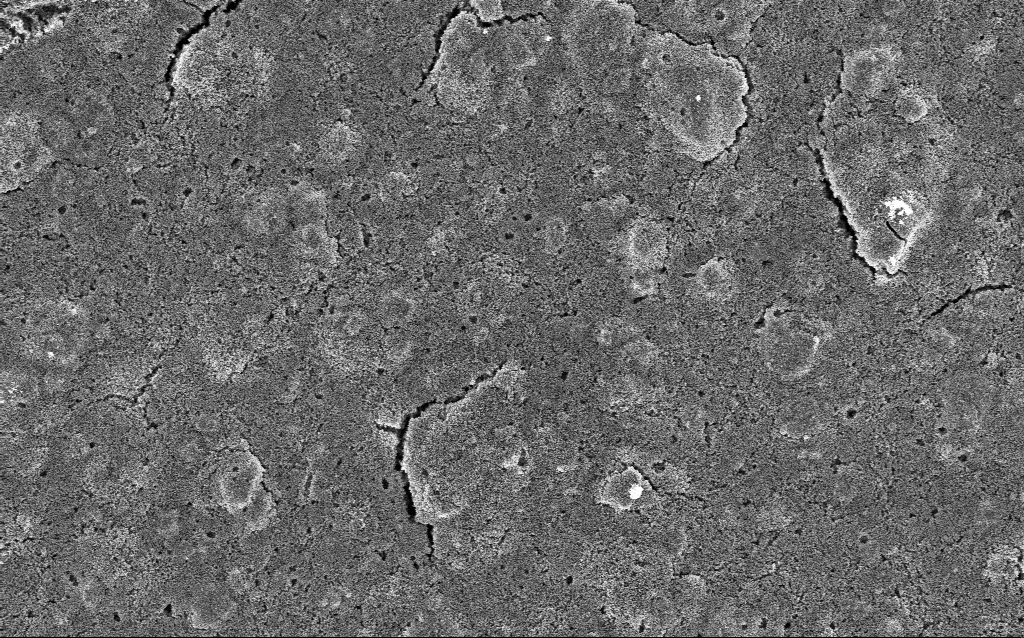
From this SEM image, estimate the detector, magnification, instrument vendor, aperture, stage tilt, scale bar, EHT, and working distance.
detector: InLens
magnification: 5 K X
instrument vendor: Zeiss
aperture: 30 µm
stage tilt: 0°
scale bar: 10000 nm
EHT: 5 kV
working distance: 2.9 mm